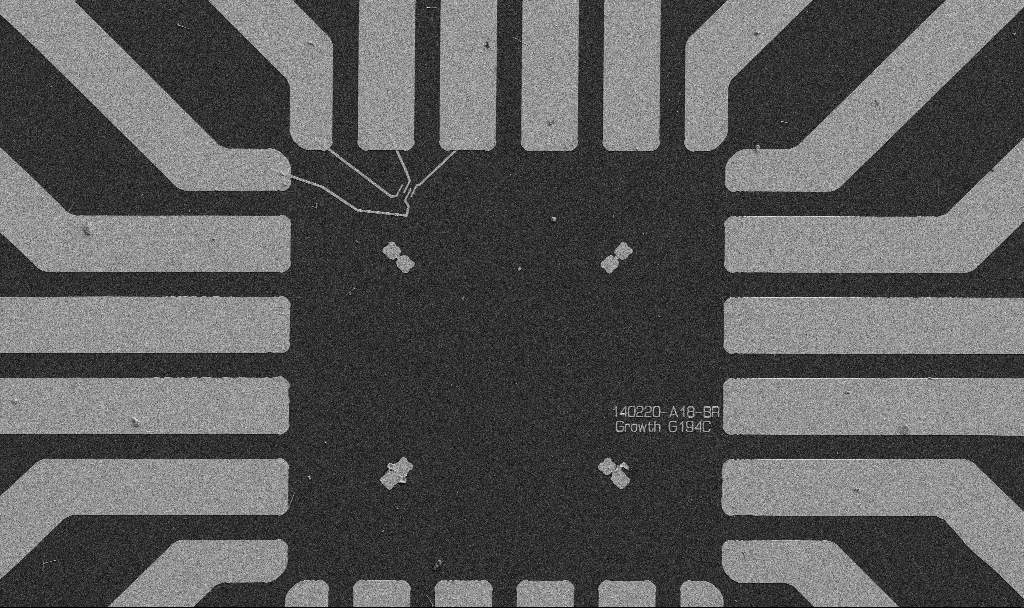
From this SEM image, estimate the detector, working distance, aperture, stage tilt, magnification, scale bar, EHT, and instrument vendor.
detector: SE2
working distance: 10.7 mm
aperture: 30 µm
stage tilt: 0°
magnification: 1 K X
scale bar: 20000 nm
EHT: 5 kV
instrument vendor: Zeiss